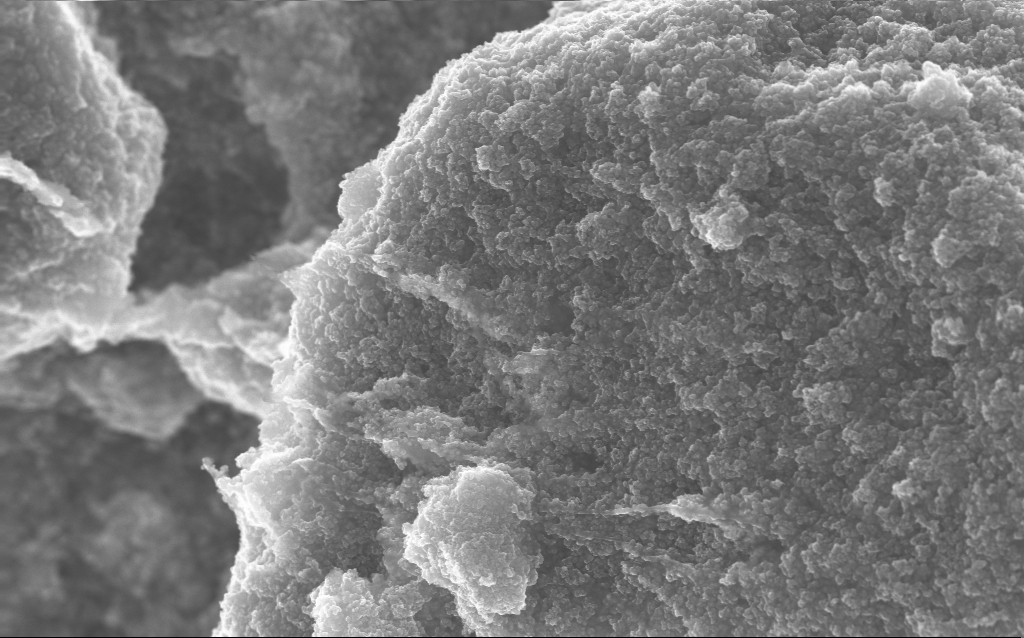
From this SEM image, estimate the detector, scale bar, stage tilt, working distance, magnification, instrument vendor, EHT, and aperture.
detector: InLens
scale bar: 1000 nm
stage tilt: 0°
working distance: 2.7 mm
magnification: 37.88 K X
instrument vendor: Zeiss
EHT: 10 kV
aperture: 30 µm